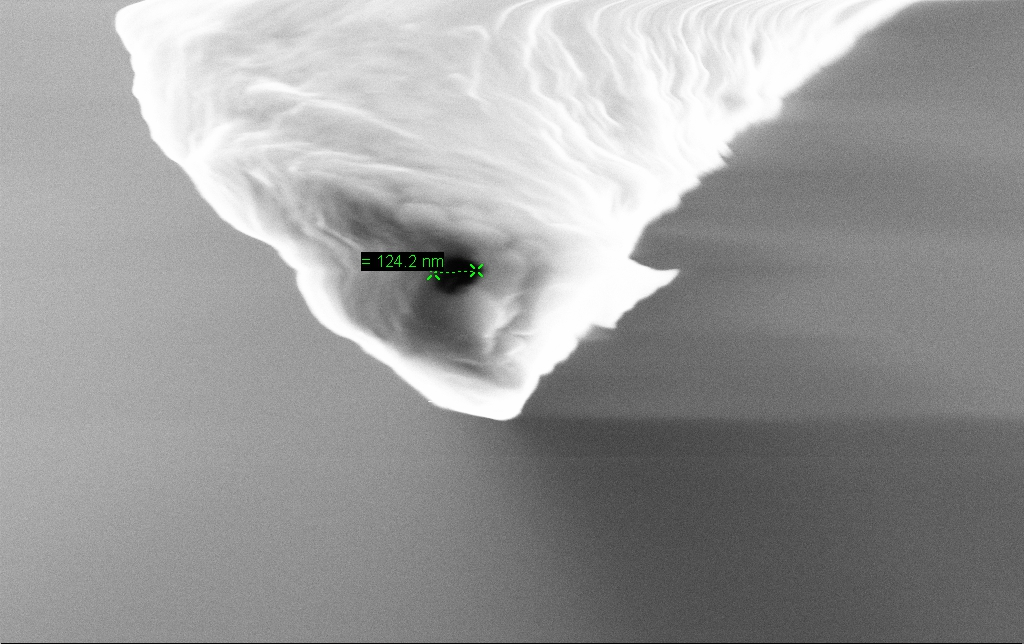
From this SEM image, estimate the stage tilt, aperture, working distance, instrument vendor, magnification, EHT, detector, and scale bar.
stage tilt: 70°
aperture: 30 µm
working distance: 7.4 mm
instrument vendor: Zeiss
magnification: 127.41 K X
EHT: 10 kV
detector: SE2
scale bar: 200 nm